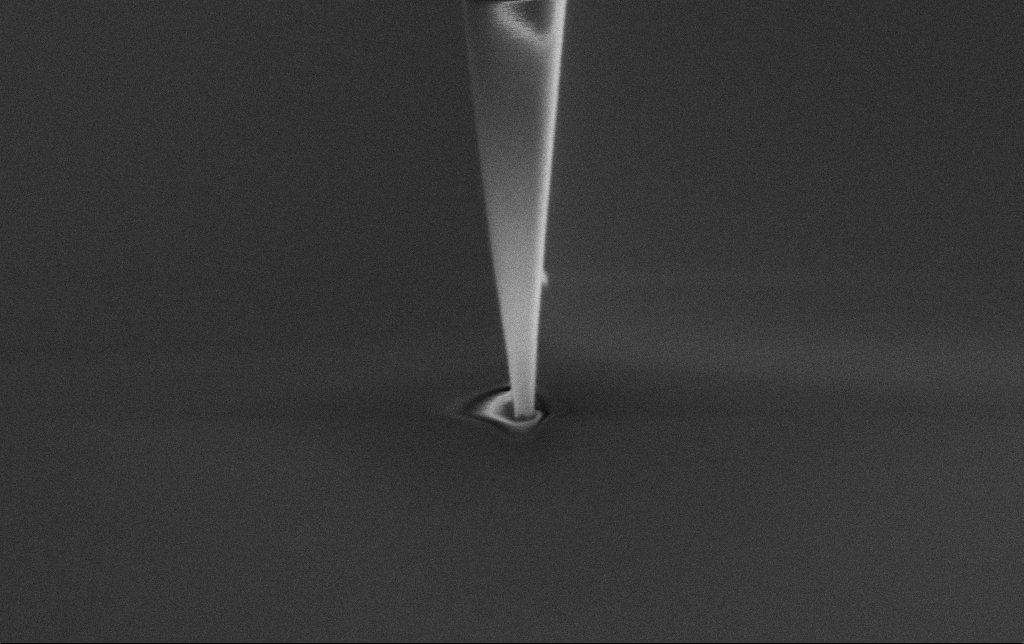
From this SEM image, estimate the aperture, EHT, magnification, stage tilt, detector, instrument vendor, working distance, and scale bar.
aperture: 30 µm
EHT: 2 kV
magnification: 100 K X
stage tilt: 0°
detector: SE2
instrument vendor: Zeiss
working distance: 6.6 mm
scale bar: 200 nm